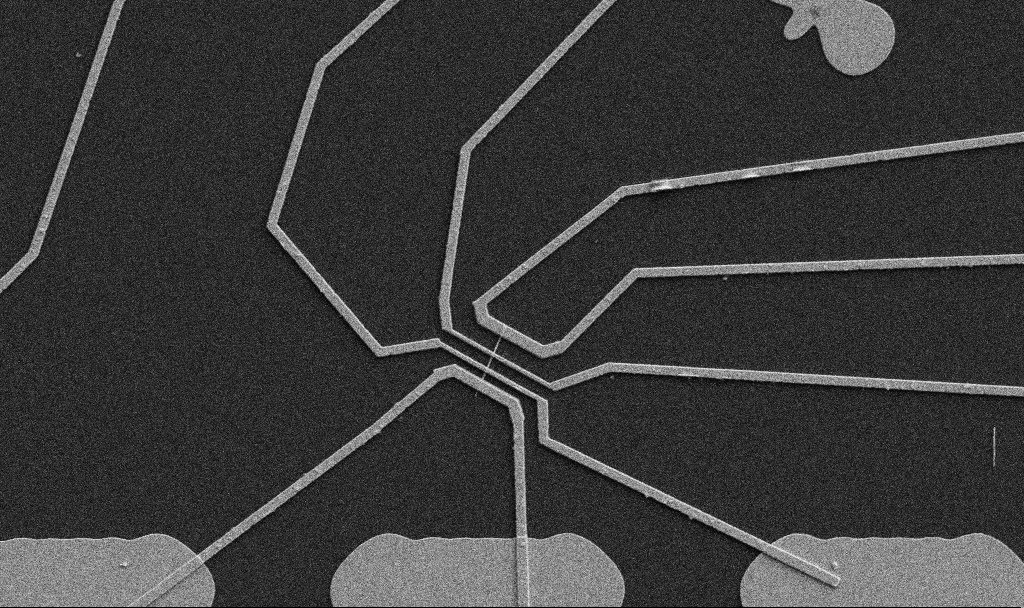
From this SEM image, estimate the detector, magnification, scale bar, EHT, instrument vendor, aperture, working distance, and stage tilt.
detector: SE2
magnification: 5 K X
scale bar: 10000 nm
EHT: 5 kV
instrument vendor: Zeiss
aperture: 30 µm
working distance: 10.7 mm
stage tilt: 0°